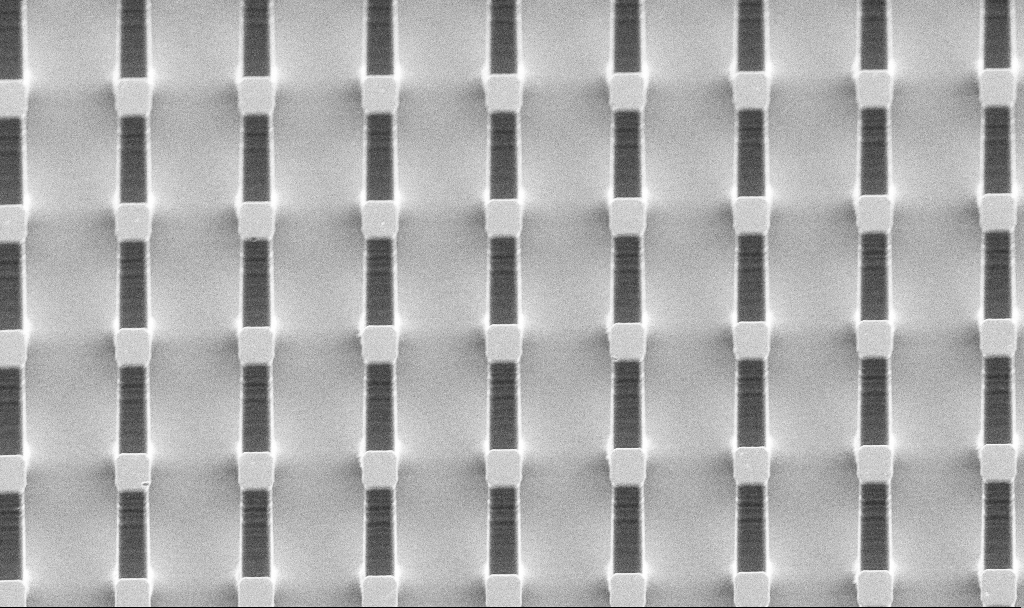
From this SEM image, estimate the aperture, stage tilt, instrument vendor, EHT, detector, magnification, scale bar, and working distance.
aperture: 30 µm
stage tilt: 45°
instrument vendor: Zeiss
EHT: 5 kV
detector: SE2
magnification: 1.52 K X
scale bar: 20000 nm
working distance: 11.7 mm